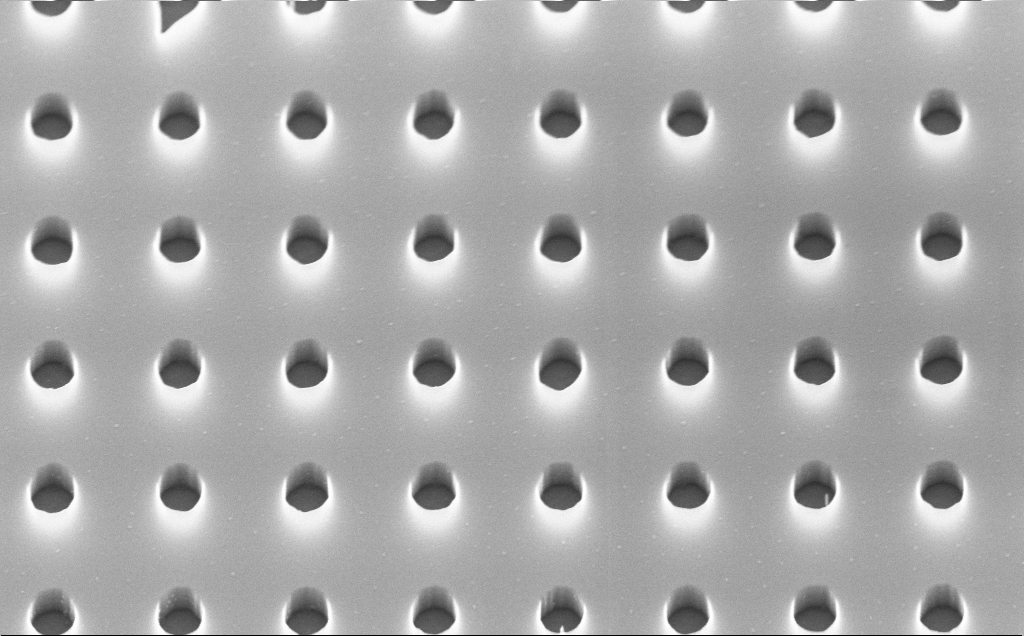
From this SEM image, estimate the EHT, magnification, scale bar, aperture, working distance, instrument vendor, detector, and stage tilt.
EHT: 10 kV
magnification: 44.69 K X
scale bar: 1000 nm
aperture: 30 µm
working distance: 4 mm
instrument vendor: Zeiss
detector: InLens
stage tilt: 45°